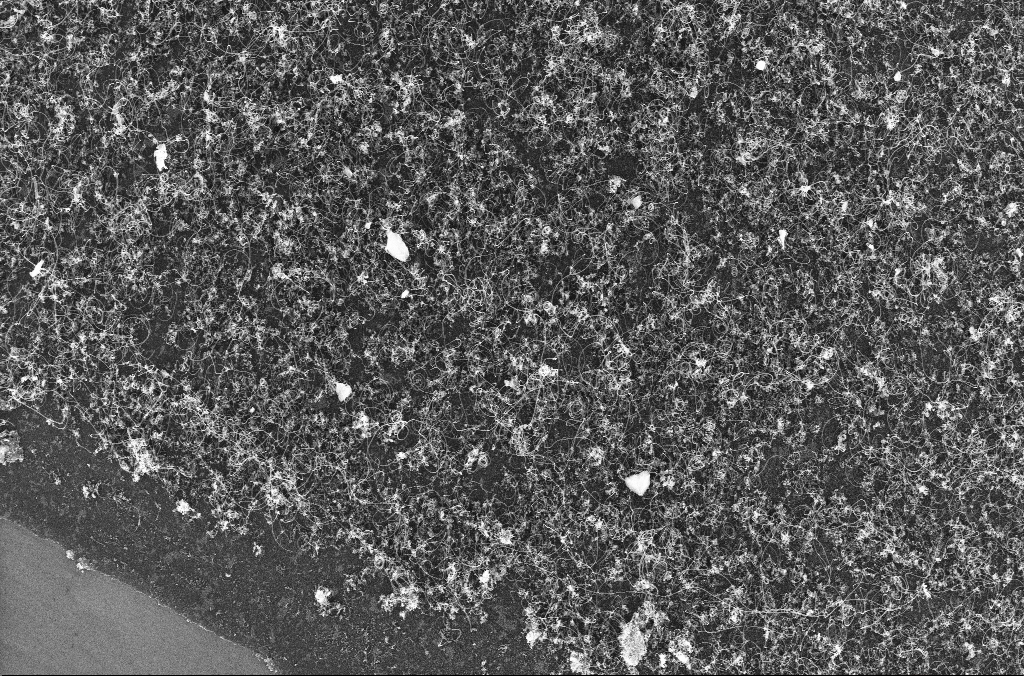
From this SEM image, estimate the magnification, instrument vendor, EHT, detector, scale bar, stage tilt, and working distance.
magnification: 2 K X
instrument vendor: Zeiss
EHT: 20 kV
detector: InLens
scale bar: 10000 nm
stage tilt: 0°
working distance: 4.2 mm